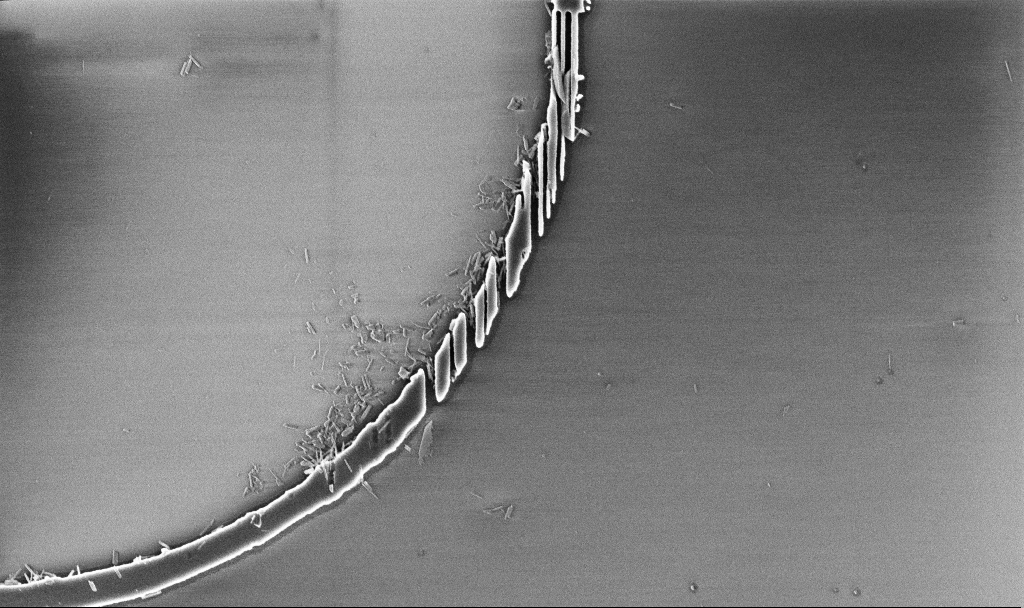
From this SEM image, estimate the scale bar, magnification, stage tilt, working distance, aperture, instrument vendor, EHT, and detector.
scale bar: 2000 nm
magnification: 22.82 K X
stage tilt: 0°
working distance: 3 mm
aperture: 30 µm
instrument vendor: Zeiss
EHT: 3 kV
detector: InLens